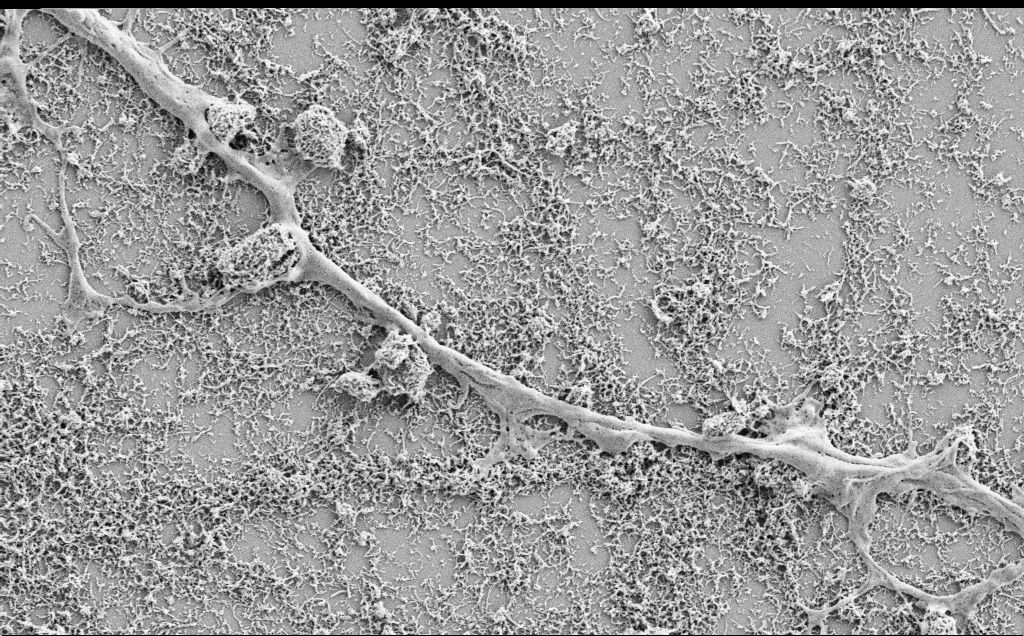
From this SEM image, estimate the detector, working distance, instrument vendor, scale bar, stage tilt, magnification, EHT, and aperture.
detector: SE2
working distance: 7.1 mm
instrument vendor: Zeiss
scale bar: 2000 nm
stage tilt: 0°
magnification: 10 K X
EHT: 2 kV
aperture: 30 µm